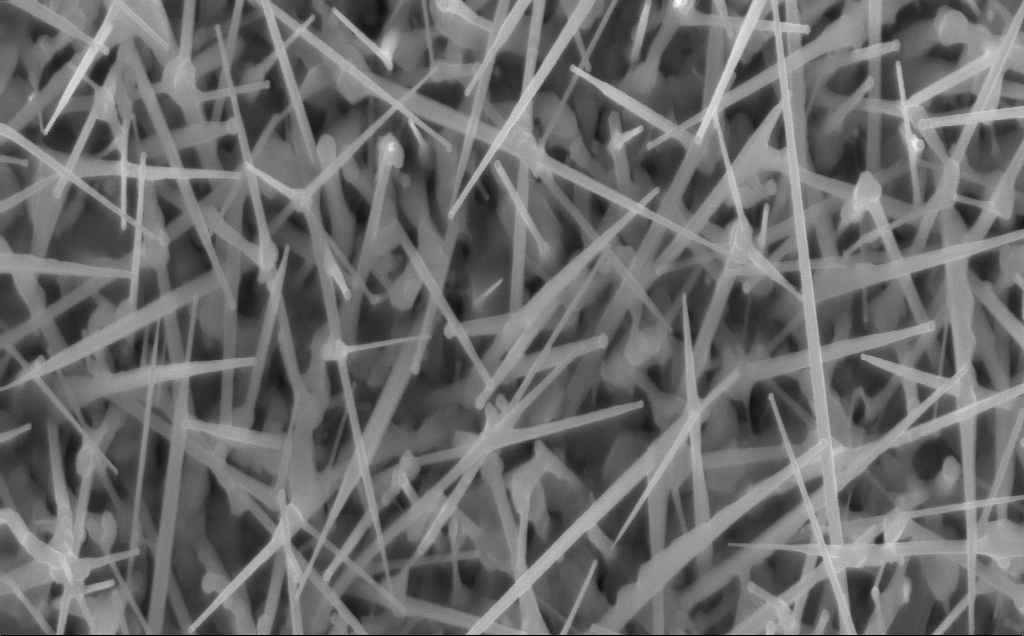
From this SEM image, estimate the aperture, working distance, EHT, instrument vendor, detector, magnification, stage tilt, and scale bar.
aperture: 30 µm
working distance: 4 mm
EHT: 10 kV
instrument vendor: Zeiss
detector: InLens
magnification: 40 K X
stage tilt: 0°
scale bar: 1000 nm